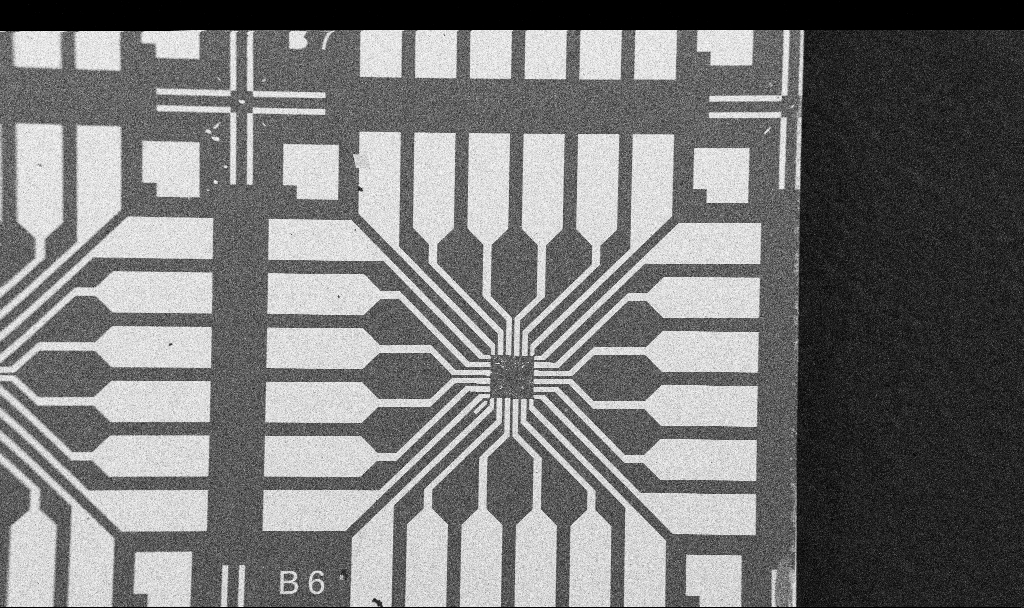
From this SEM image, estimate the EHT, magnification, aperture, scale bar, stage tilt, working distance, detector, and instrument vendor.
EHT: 5 kV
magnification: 0.1 K X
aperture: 30 µm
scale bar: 200000 nm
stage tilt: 0°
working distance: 10.7 mm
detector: SE2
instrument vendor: Zeiss